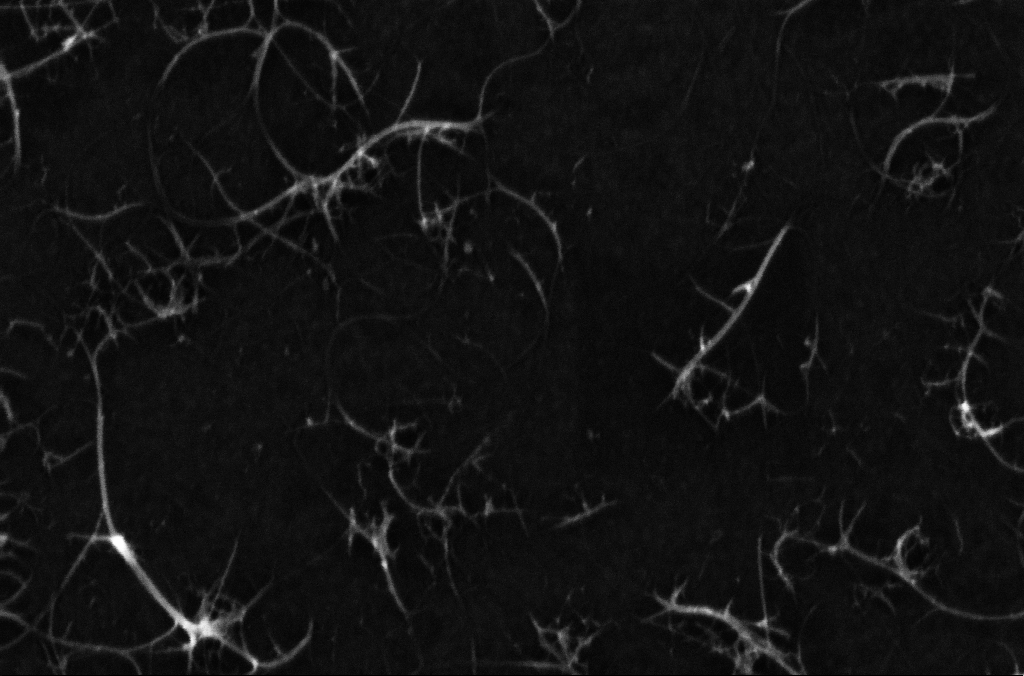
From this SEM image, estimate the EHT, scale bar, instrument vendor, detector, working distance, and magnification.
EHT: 10 kV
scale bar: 200 nm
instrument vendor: Zeiss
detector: InLens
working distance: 3.2 mm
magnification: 272.29 K X